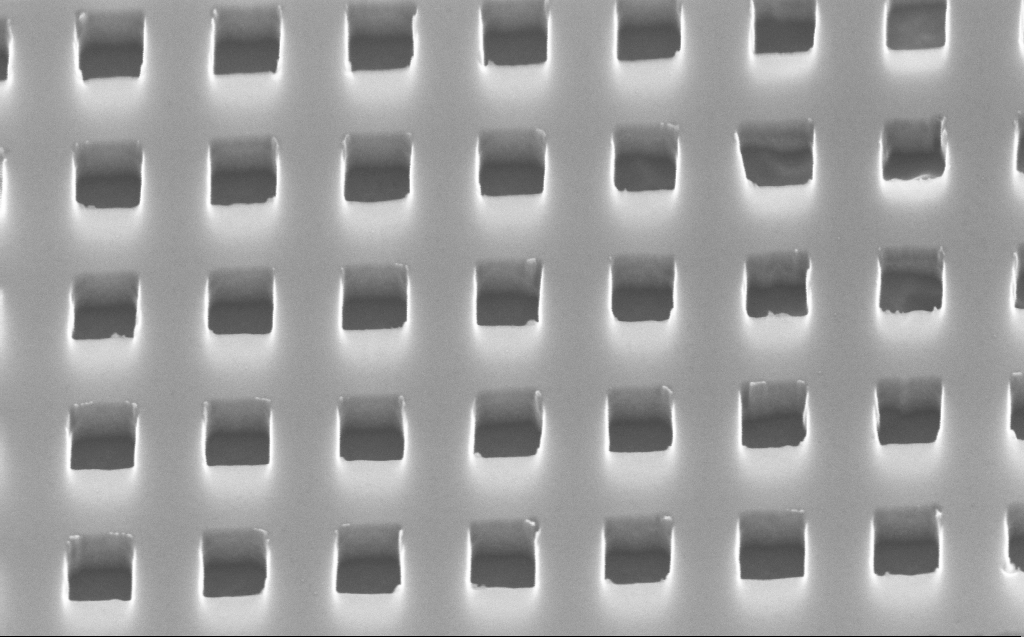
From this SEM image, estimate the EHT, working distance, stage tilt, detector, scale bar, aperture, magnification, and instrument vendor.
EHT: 10 kV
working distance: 3 mm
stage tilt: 45°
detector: InLens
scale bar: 200 nm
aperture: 30 µm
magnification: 100 K X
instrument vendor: Zeiss